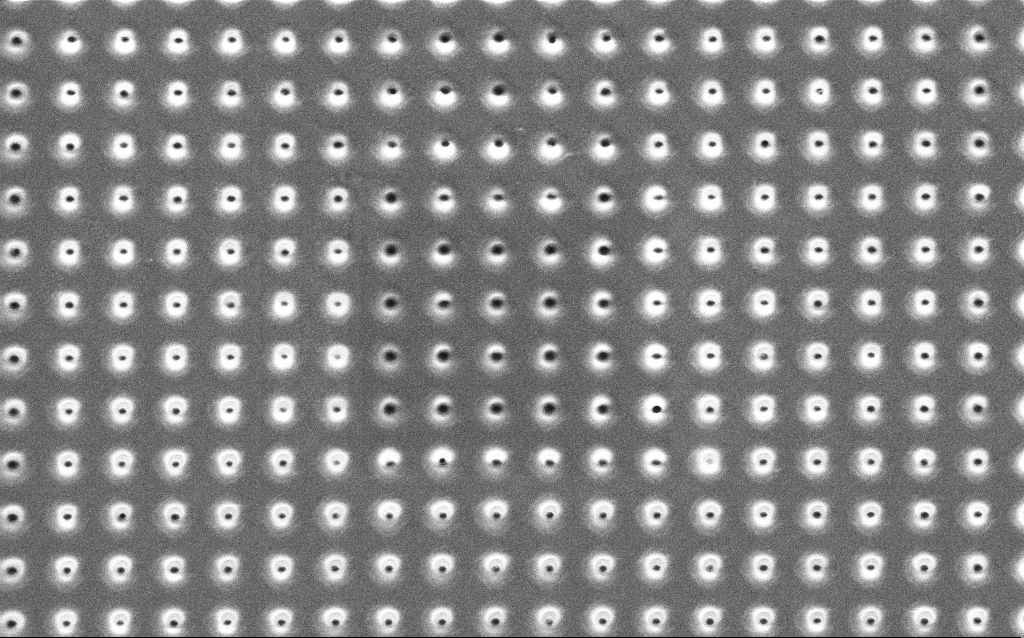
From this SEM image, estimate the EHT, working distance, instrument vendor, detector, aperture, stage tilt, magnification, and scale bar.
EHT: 1.5 kV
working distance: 6.7 mm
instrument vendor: Zeiss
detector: SE2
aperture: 30 µm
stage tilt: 0°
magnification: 18.69 K X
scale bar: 2000 nm